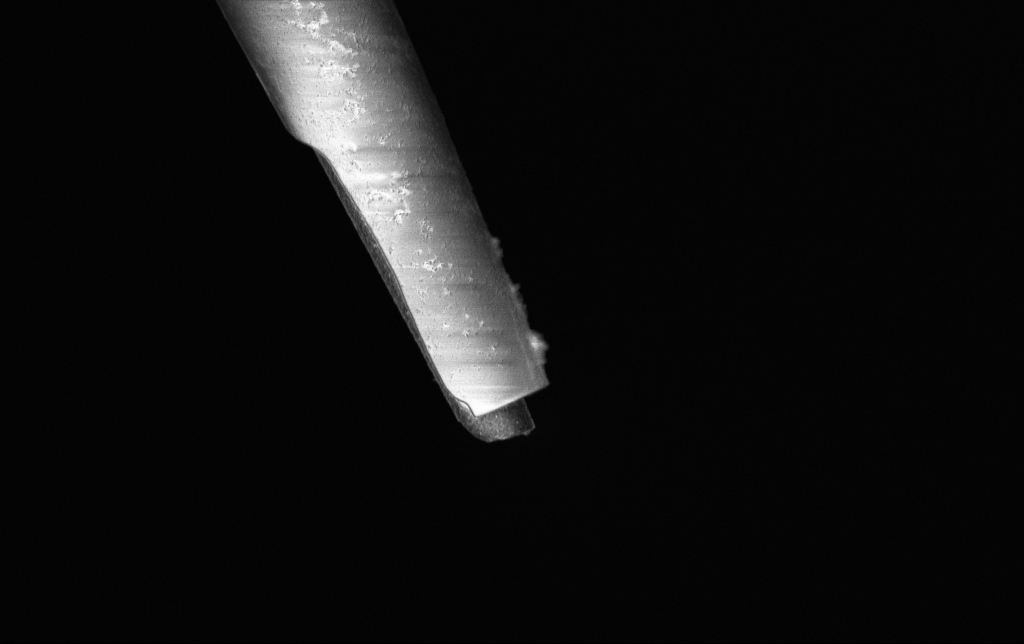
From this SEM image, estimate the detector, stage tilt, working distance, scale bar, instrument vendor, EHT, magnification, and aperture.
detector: InLens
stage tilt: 0°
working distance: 6.5 mm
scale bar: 2000 nm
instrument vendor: Zeiss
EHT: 3 kV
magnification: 10 K X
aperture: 30 µm